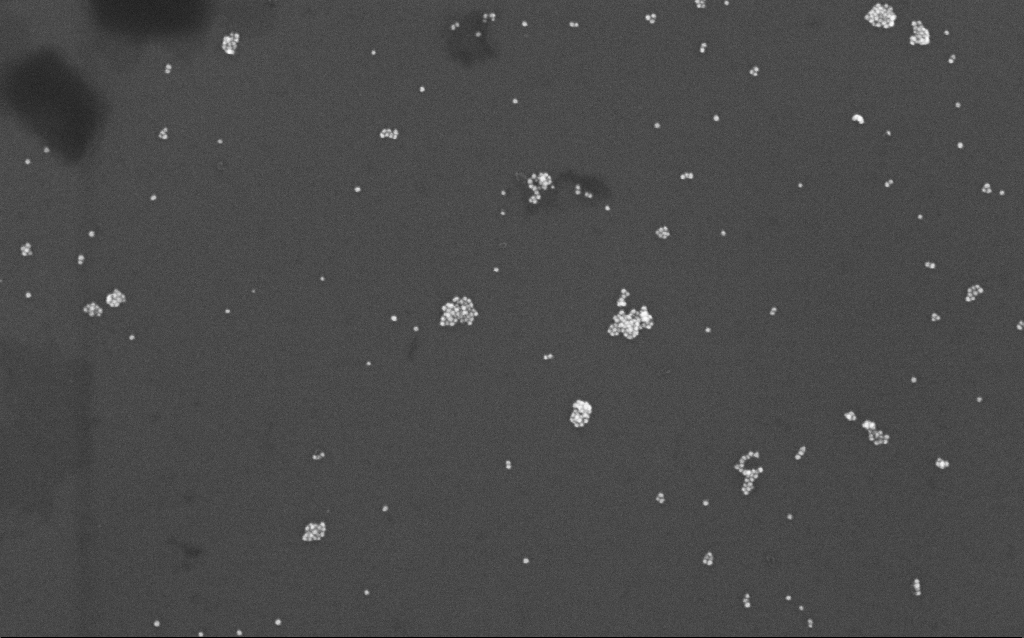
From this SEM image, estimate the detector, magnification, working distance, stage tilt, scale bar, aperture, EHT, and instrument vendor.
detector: InLens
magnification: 96.05 K X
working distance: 7 mm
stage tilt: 0°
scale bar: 200 nm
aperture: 30 µm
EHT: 10 kV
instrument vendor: Zeiss